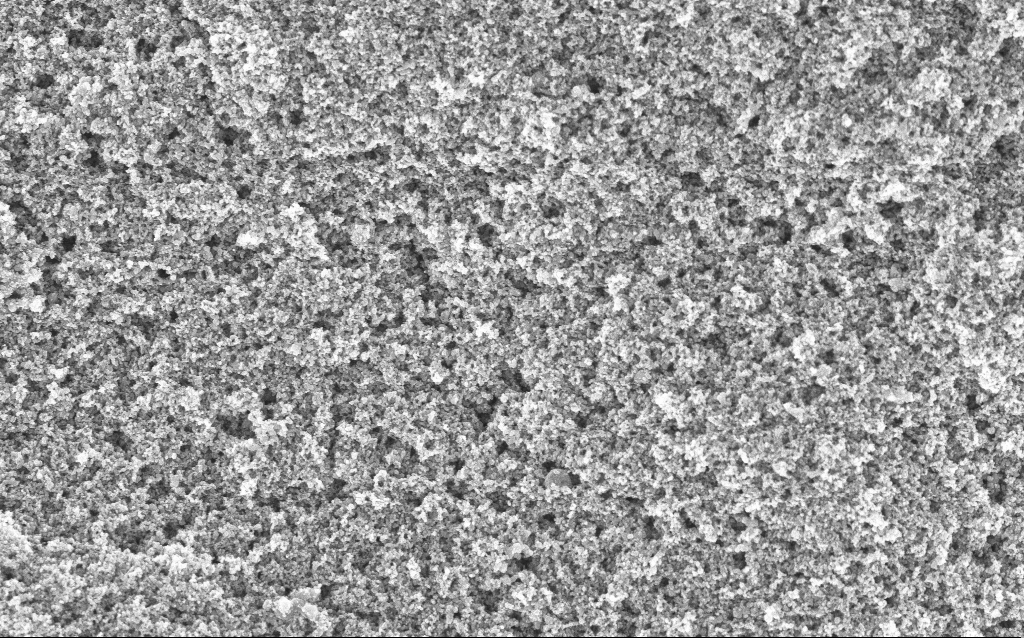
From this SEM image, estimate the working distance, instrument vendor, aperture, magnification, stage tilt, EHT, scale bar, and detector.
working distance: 4.4 mm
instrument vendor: Zeiss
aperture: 30 µm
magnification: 37.88 K X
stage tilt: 0°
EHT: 5 kV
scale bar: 1000 nm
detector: InLens